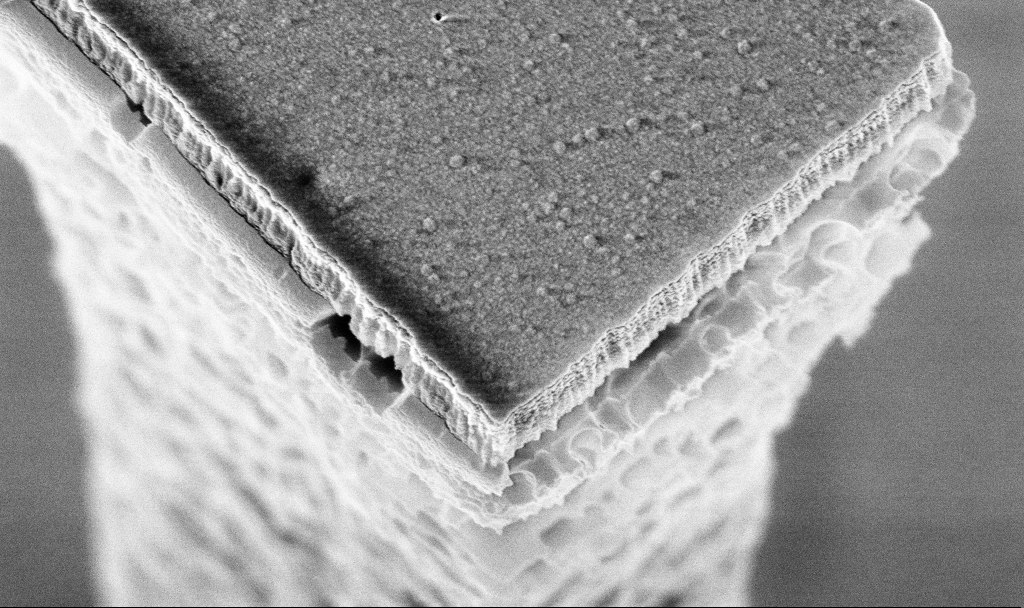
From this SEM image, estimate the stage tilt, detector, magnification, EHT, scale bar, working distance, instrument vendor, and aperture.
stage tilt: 20°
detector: InLens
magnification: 76.59 K X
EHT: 5 kV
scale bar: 200 nm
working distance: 3.3 mm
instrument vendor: Zeiss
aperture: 30 µm